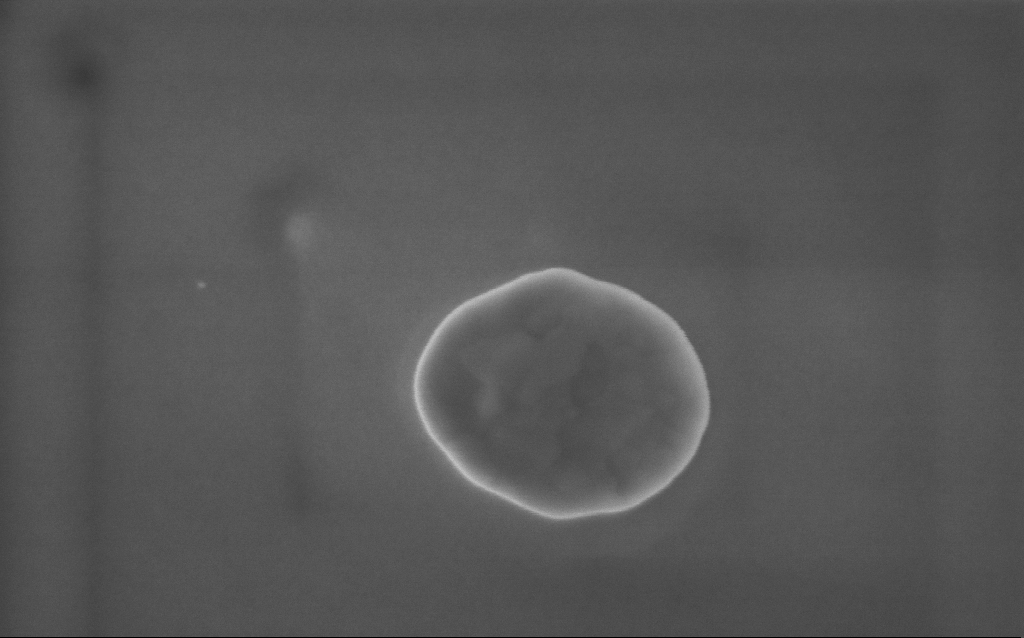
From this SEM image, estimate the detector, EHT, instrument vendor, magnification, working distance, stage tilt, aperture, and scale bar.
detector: InLens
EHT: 5 kV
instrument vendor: Zeiss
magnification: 118 K X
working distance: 4 mm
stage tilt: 0°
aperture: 30 µm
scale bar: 200 nm